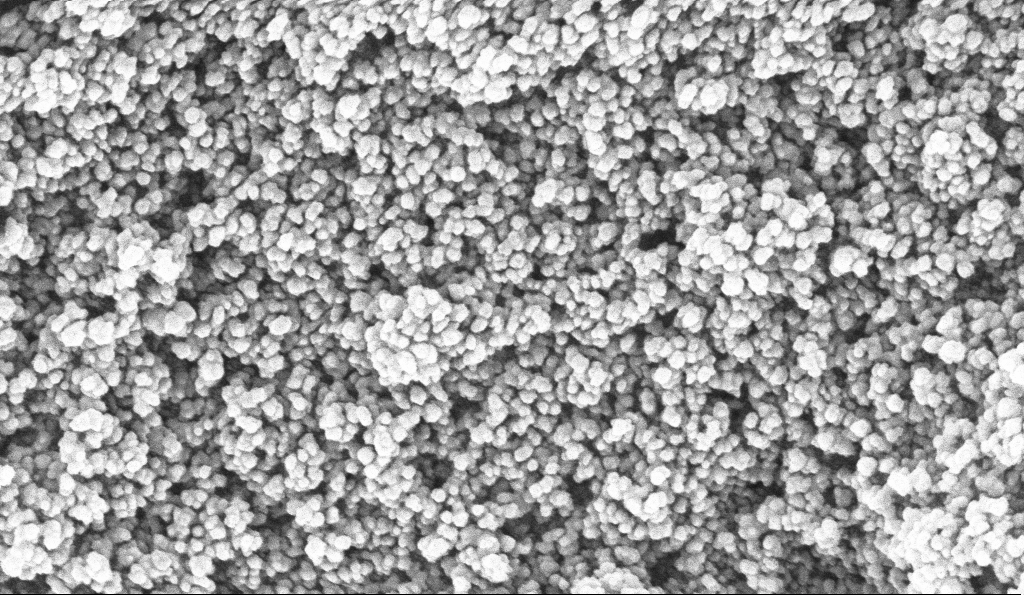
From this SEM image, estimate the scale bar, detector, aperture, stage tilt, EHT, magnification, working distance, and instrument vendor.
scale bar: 100 nm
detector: InLens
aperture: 30 µm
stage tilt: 0°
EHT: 10 kV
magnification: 135 K X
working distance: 5.1 mm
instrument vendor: Zeiss